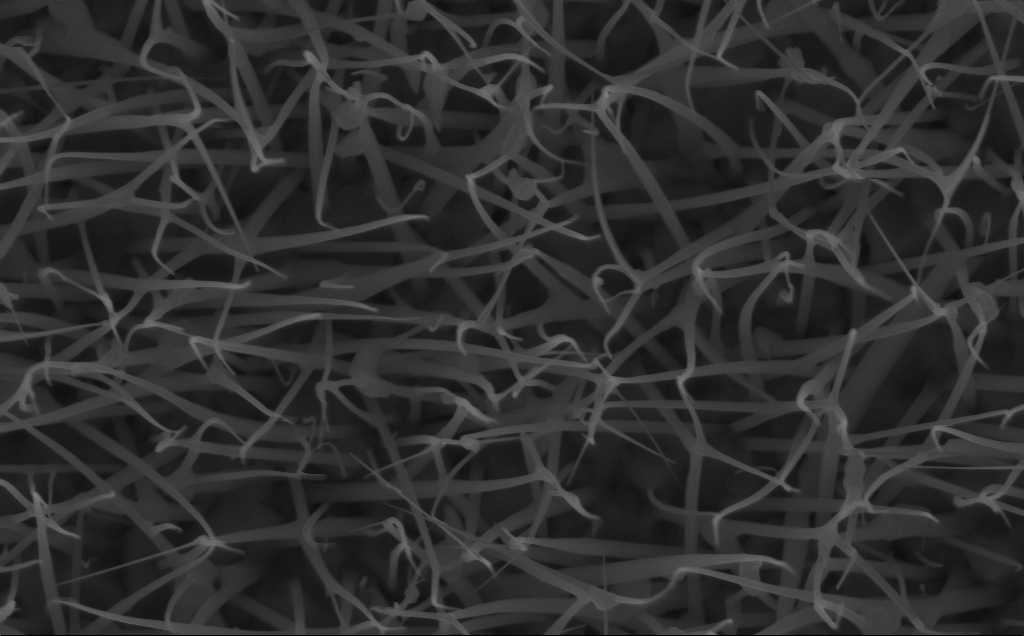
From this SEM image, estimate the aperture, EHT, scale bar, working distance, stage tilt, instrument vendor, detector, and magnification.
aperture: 30 µm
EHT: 10 kV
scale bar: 1000 nm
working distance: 6 mm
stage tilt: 0°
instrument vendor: Zeiss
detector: InLens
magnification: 40 K X